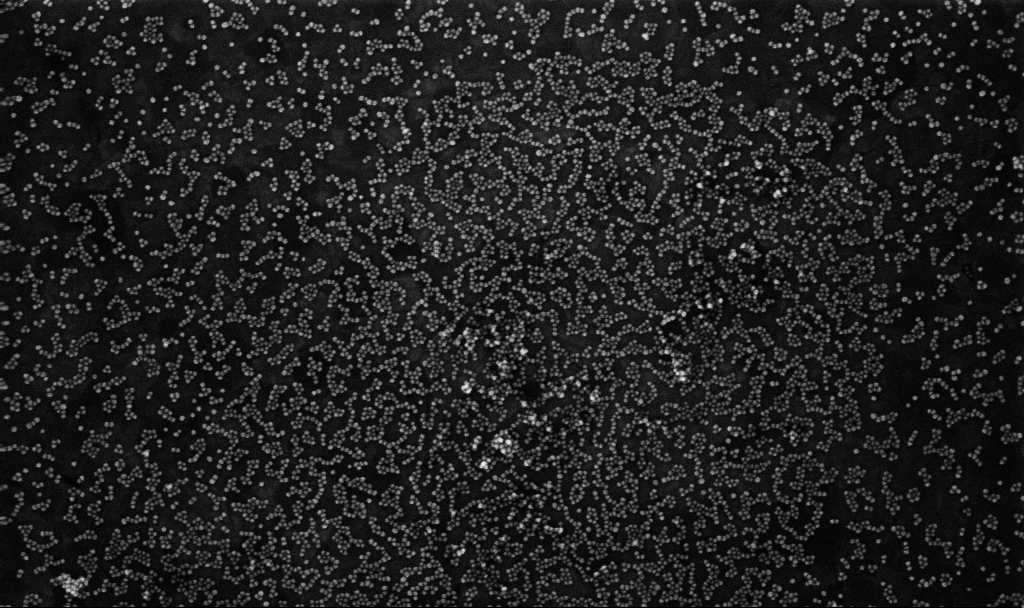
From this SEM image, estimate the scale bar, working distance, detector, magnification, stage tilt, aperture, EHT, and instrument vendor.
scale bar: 200 nm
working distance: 3.8 mm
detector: InLens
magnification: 100 K X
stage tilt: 0°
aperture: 30 µm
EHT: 10 kV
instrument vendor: Zeiss